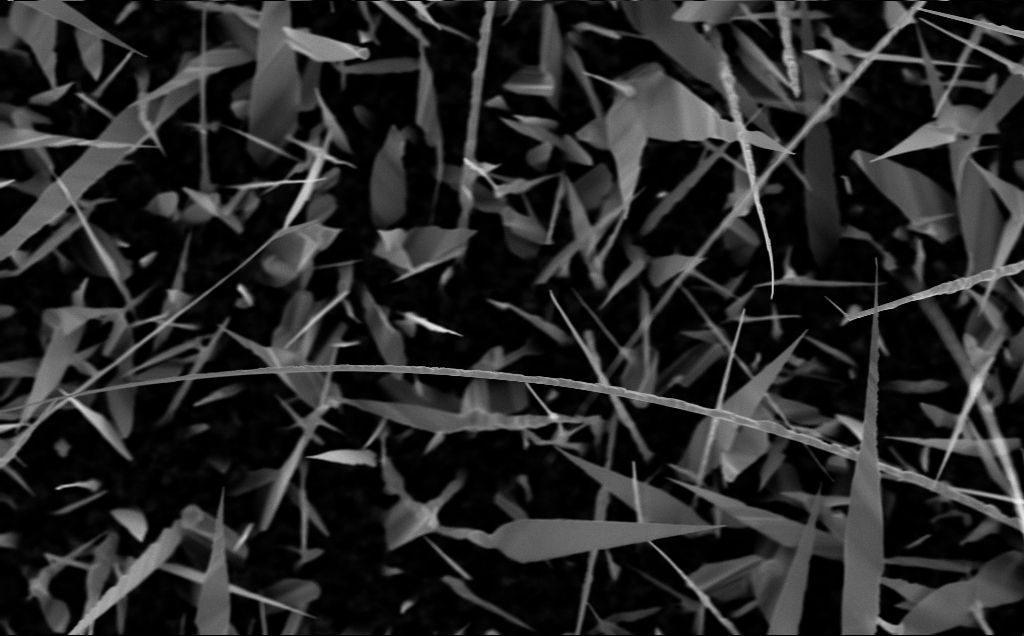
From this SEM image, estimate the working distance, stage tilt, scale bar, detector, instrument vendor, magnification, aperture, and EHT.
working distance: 6 mm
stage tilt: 0°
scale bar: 1000 nm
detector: InLens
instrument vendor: Zeiss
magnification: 20 K X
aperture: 30 µm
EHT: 10 kV